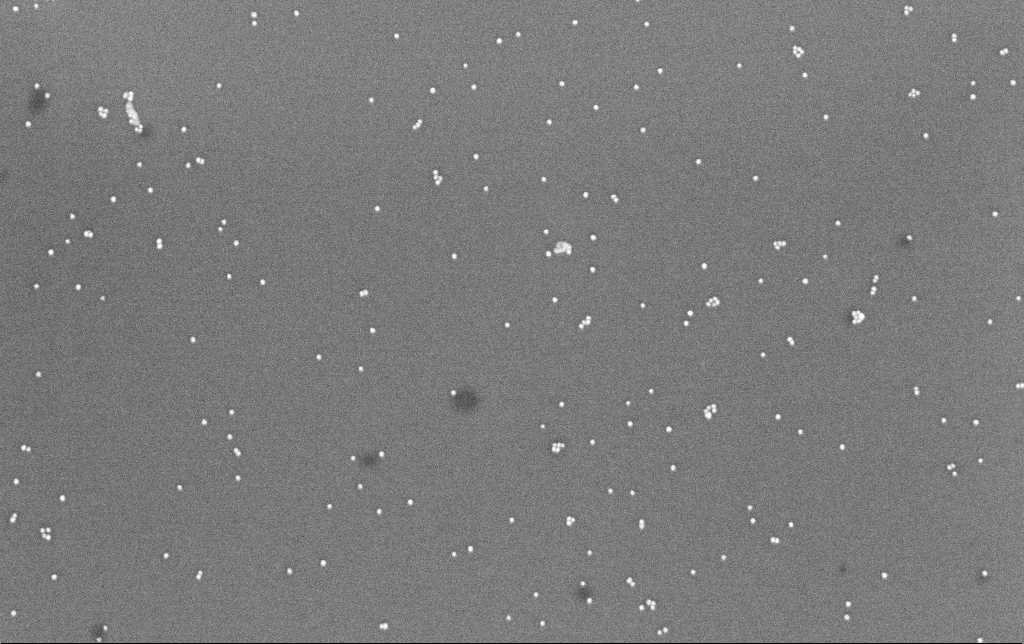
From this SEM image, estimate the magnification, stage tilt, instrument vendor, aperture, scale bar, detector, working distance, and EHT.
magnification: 100 K X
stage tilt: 0°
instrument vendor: Zeiss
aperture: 30 µm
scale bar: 200 nm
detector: InLens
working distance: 6.6 mm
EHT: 8 kV